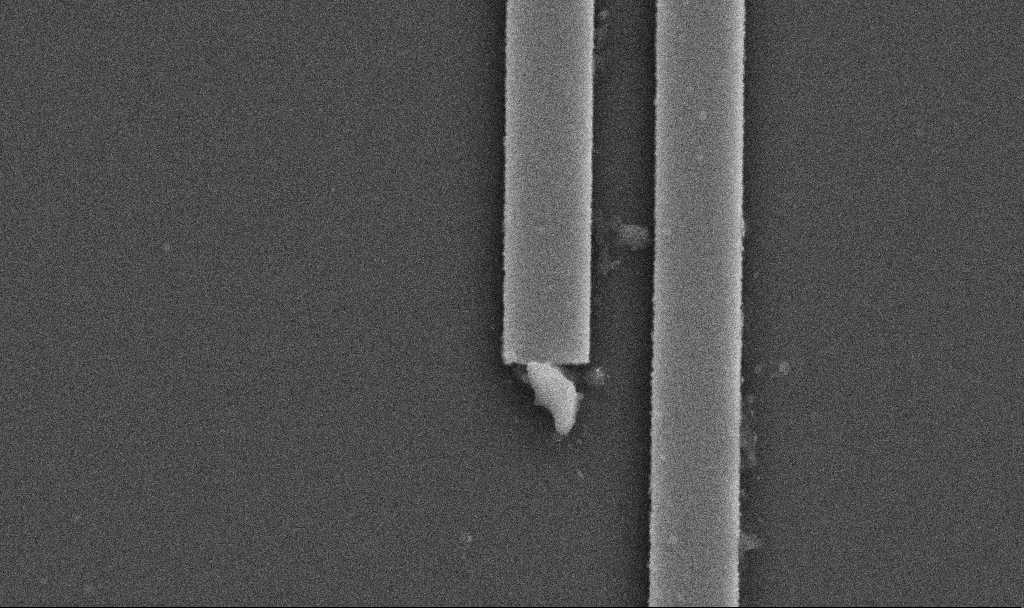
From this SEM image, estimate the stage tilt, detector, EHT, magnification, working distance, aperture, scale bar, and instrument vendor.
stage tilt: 0°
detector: SE2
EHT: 5 kV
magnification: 64.22 K X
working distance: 10.3 mm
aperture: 30 µm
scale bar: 1000 nm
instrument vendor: Zeiss